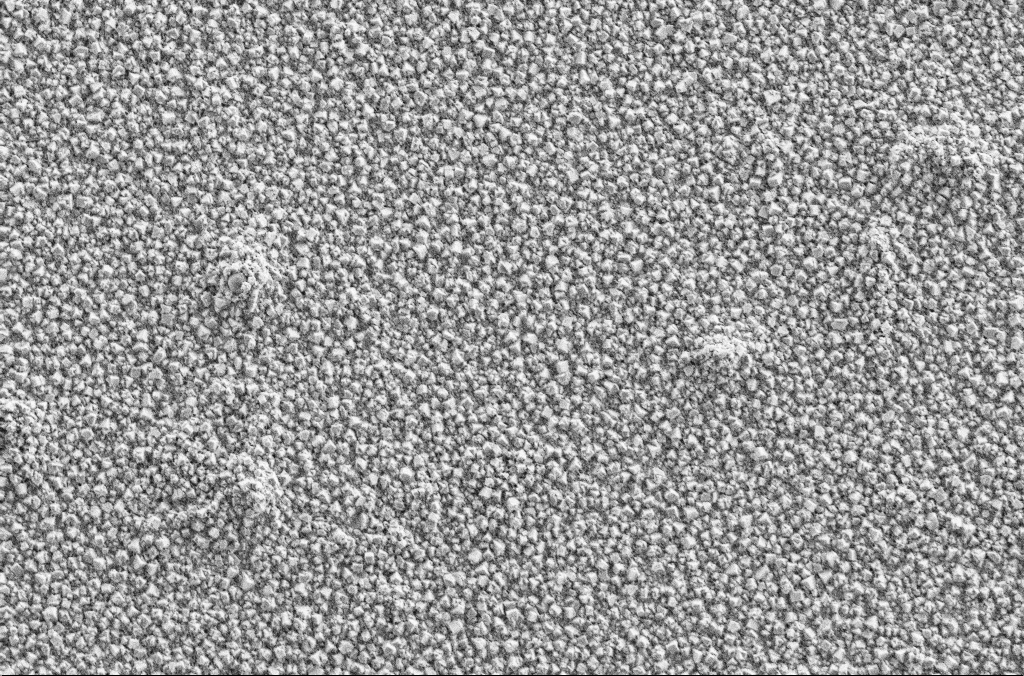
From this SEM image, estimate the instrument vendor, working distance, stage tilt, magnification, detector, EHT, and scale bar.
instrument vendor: Zeiss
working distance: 1.9 mm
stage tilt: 0°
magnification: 10 K X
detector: SE2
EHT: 2 kV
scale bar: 2000 nm